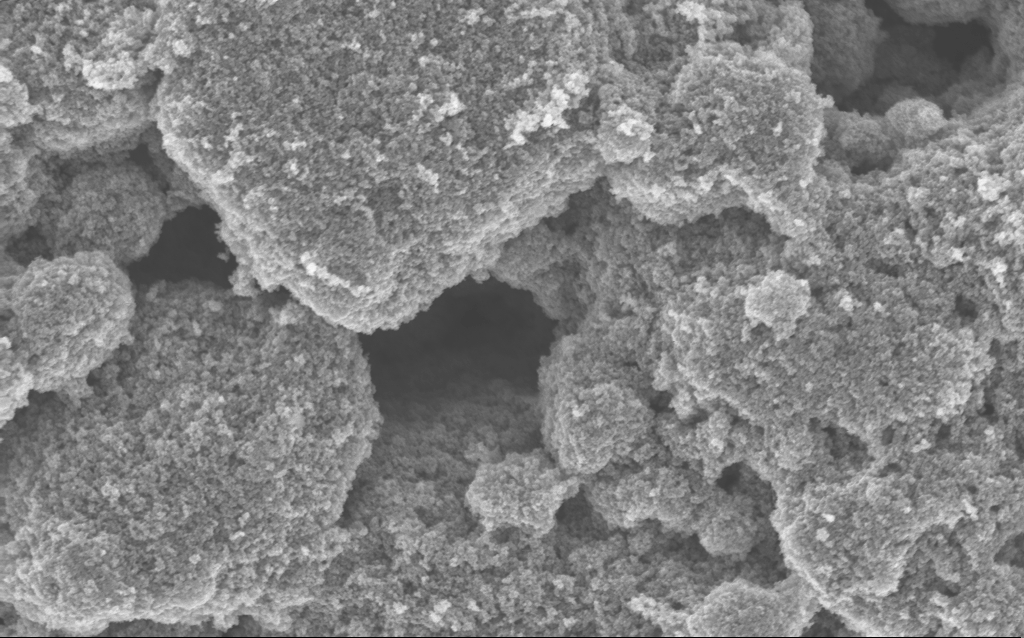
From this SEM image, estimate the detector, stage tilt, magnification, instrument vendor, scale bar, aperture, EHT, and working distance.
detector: InLens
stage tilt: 0°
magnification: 37.64 K X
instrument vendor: Zeiss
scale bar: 1000 nm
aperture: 30 µm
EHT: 5 kV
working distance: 4.7 mm